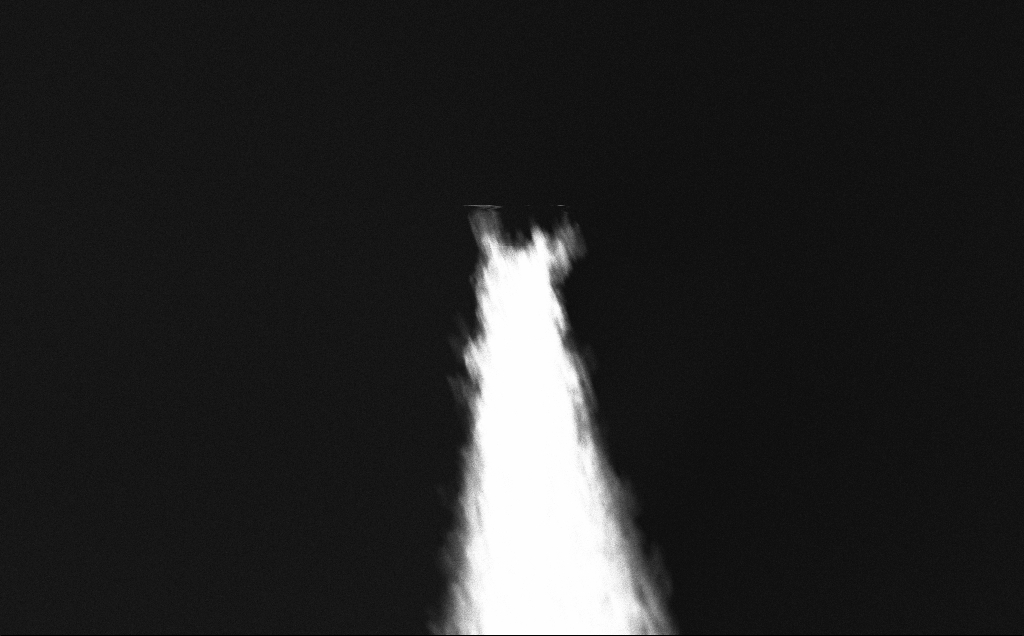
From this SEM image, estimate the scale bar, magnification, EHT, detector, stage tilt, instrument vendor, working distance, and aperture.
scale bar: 10000 nm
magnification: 5.69 K X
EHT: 5 kV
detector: InLens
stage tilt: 20°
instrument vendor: Zeiss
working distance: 3 mm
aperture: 30 µm